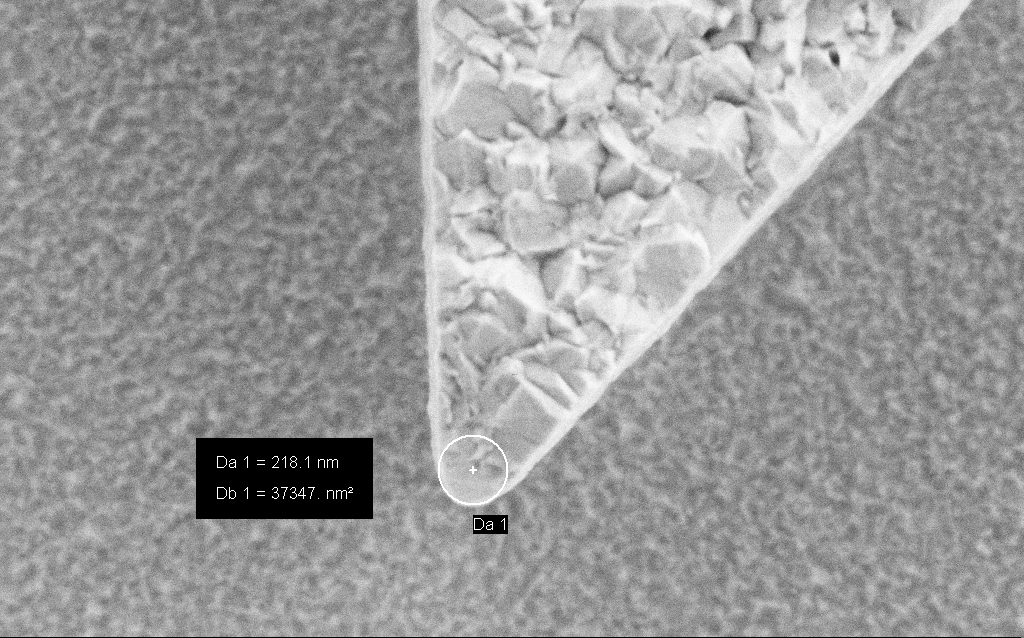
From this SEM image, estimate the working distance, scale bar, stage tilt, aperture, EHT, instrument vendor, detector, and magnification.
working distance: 4.5 mm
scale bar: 200 nm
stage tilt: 0°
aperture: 30 µm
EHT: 3 kV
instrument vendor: Zeiss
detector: SE2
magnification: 117.87 K X